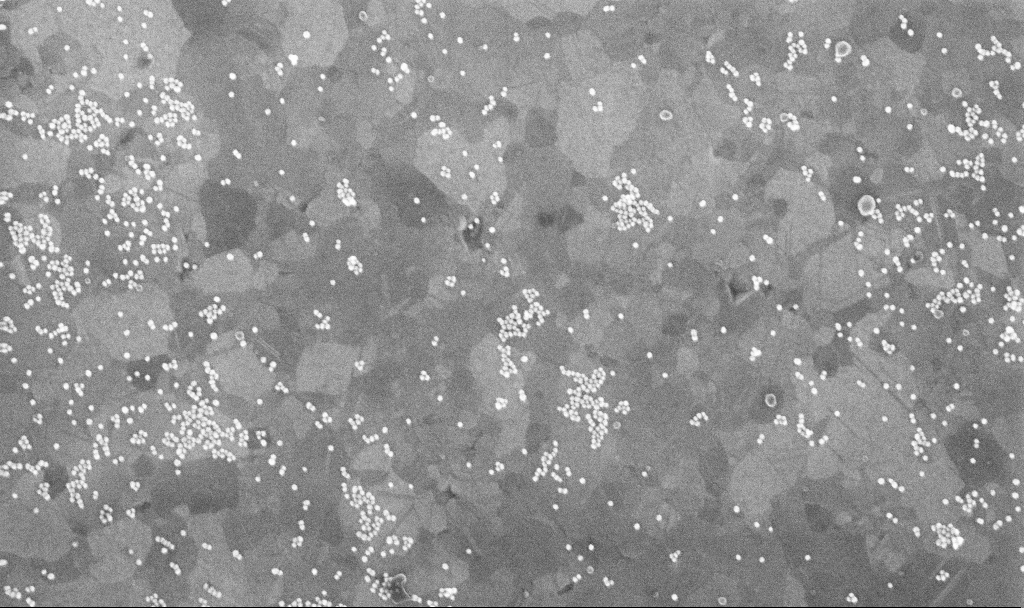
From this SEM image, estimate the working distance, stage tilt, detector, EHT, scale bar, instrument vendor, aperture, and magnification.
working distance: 3.7 mm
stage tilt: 0°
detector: InLens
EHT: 10 kV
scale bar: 200 nm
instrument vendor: Zeiss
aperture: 30 µm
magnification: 100 K X